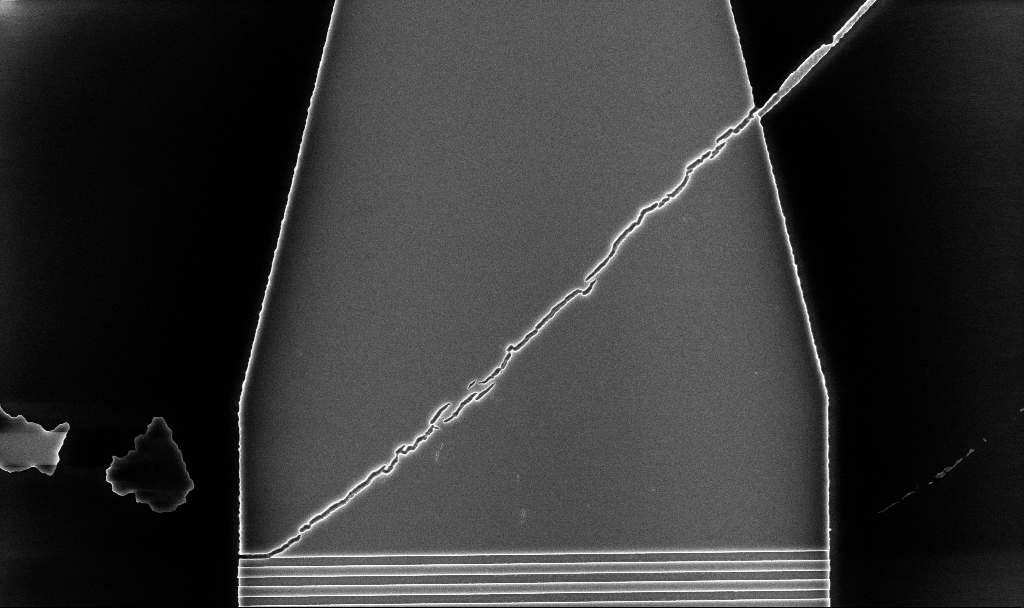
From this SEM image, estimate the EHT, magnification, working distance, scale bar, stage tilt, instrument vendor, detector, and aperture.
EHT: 5 kV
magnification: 11 K X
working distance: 5.2 mm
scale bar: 2000 nm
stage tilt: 0°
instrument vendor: Zeiss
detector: InLens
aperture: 30 µm